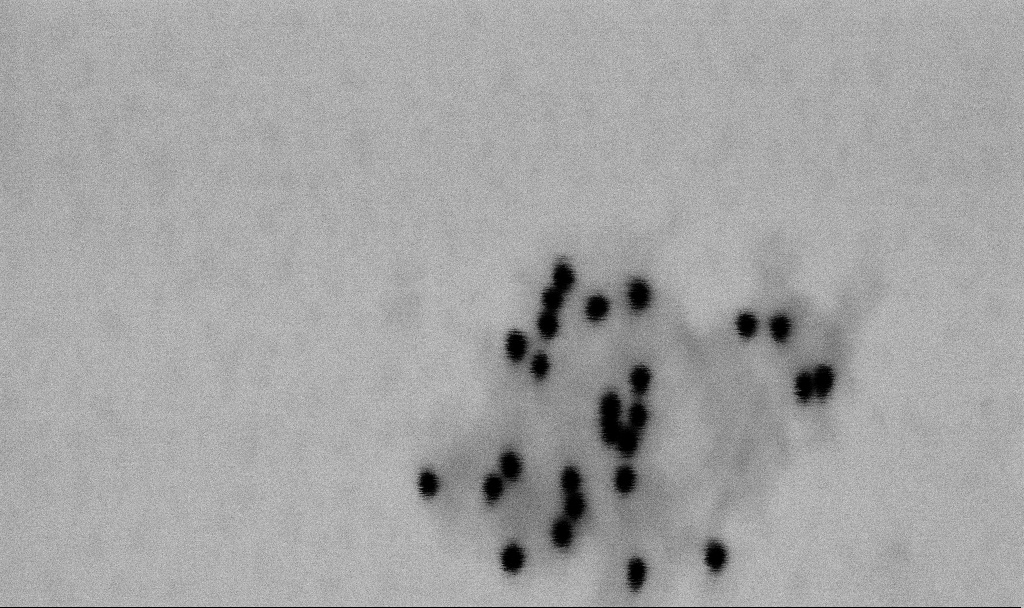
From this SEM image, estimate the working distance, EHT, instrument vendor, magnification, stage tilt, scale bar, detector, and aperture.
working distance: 6.6 mm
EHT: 2 kV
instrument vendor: Zeiss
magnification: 300 K X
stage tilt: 0°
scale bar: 100 nm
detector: SE2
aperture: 30 µm